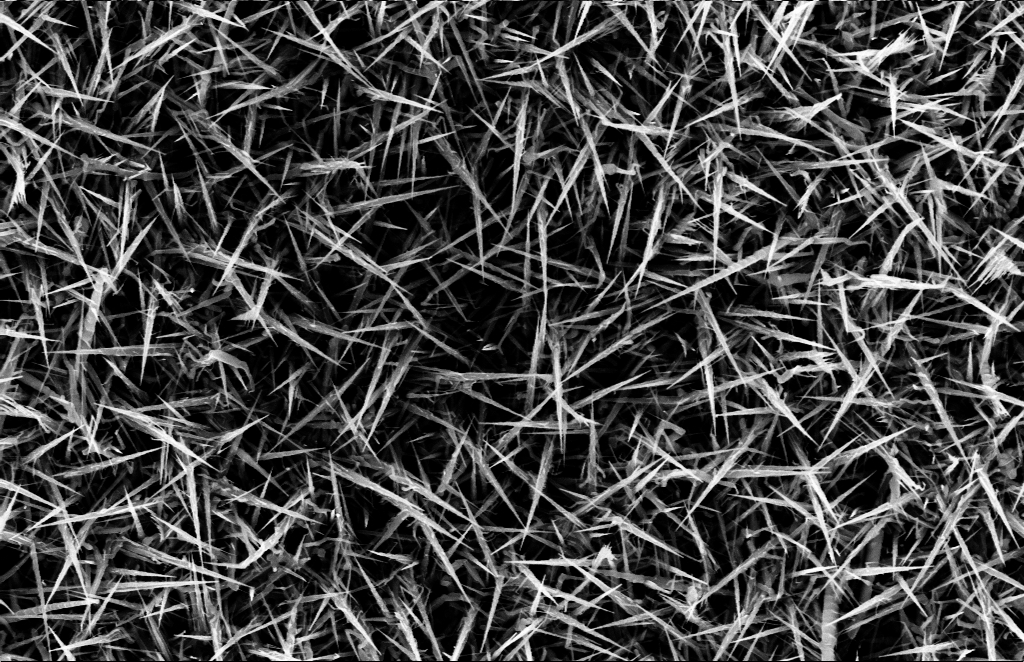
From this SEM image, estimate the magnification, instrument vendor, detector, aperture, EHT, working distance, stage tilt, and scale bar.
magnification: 40 K X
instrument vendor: Zeiss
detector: InLens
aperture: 30 µm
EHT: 10 kV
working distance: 10 mm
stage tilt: -1.1°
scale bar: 1000 nm